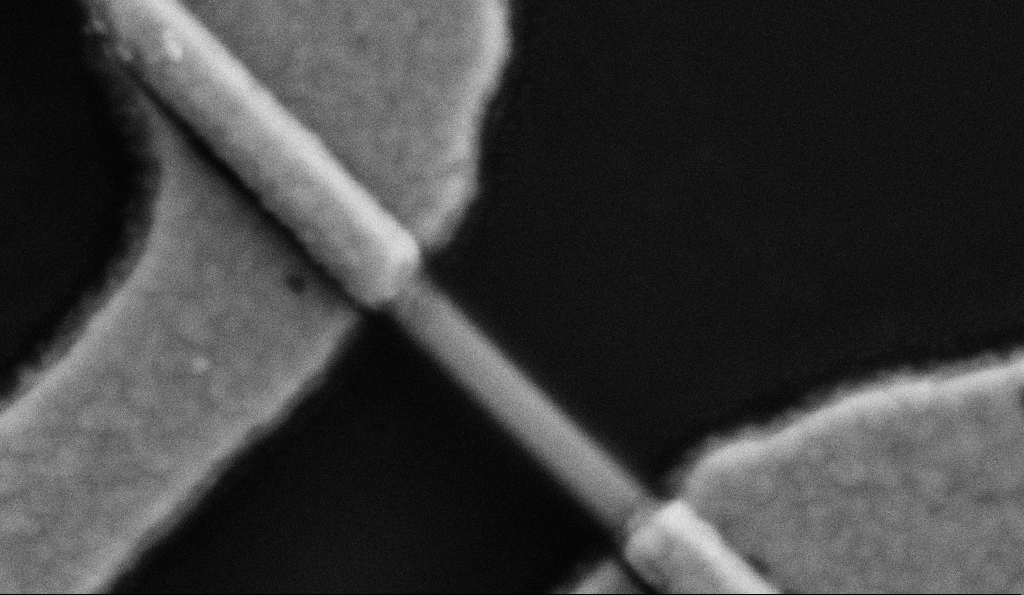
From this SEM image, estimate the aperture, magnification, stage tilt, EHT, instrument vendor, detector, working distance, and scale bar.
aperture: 30 µm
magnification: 200 K X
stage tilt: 0°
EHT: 5 kV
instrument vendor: Zeiss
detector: SE2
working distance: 8.5 mm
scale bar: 200 nm